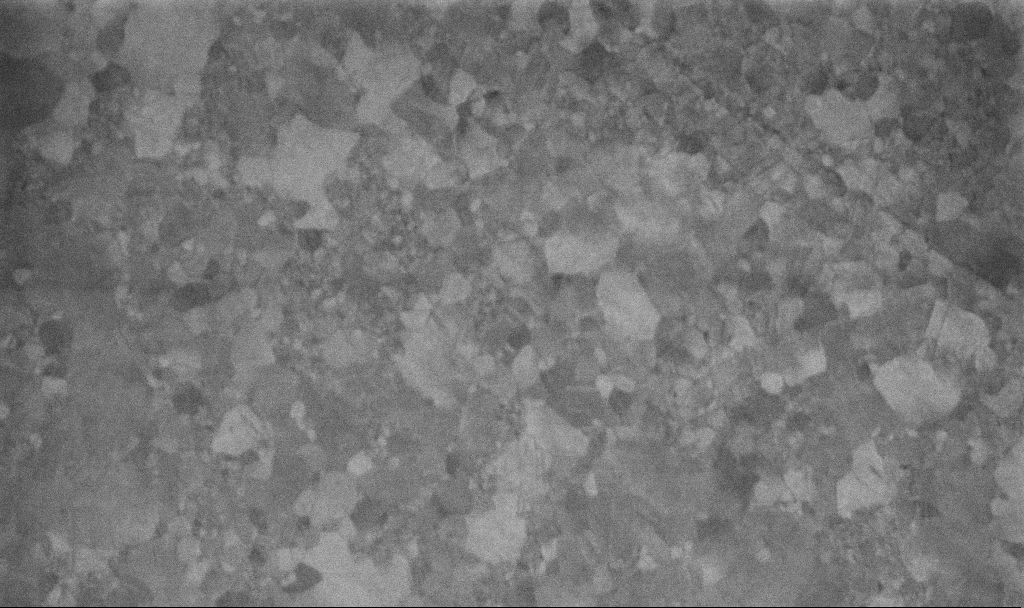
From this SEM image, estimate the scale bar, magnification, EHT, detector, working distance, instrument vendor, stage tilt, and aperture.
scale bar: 200 nm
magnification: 100 K X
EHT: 10 kV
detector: InLens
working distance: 3.4 mm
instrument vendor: Zeiss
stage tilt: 0°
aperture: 30 µm